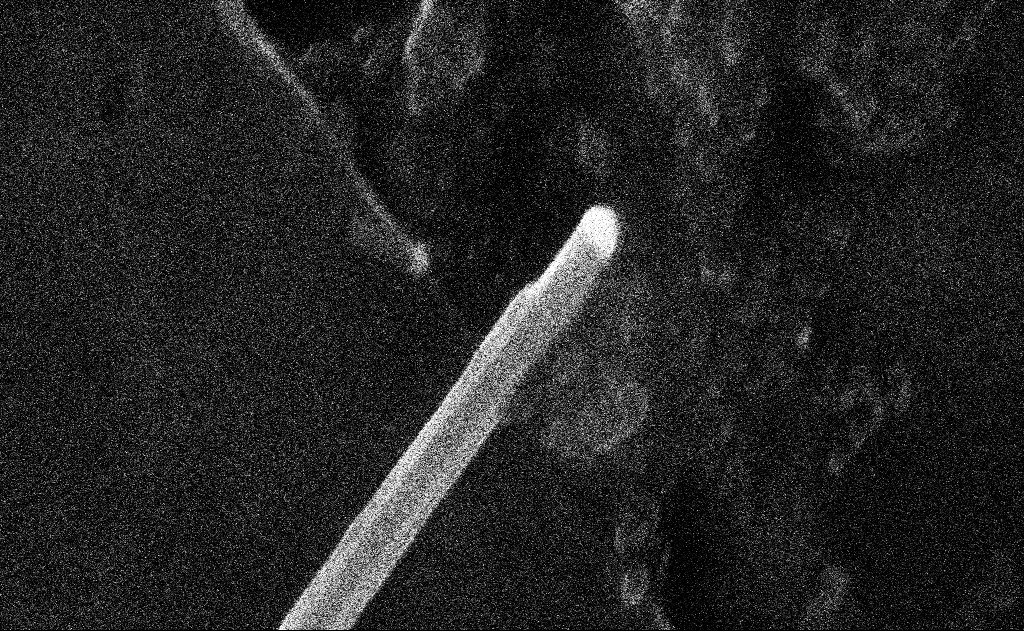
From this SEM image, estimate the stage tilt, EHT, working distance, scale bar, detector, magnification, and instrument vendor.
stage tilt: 0°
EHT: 20 kV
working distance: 11 mm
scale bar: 200 nm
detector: InLens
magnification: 230.02 K X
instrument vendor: Zeiss